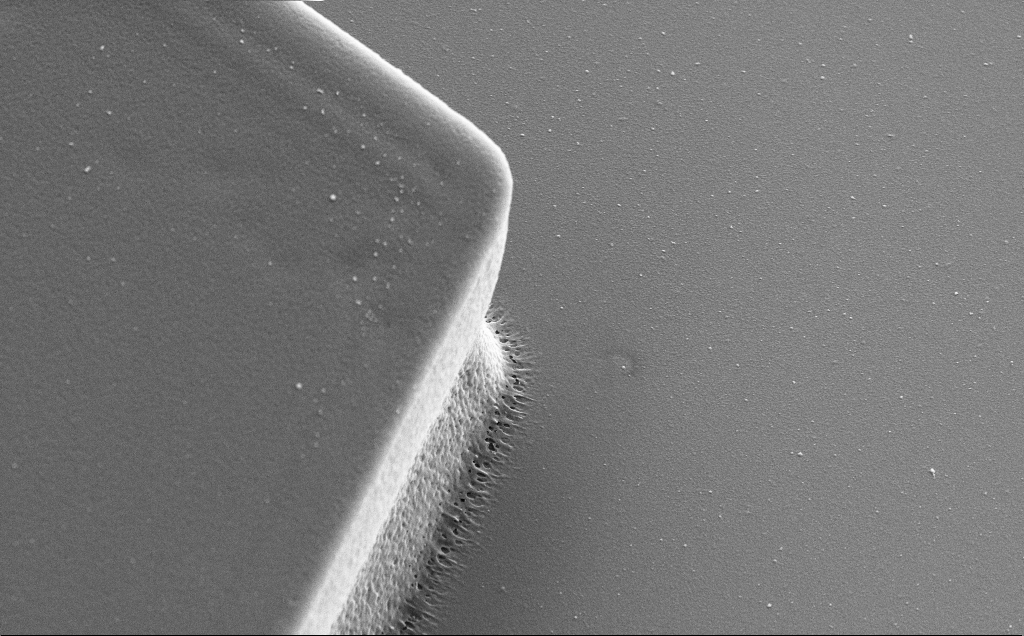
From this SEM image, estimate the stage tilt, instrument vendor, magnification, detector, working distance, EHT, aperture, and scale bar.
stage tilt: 30°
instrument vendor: Zeiss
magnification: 14.93 K X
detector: SE2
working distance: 11 mm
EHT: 5 kV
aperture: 30 µm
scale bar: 1000 nm